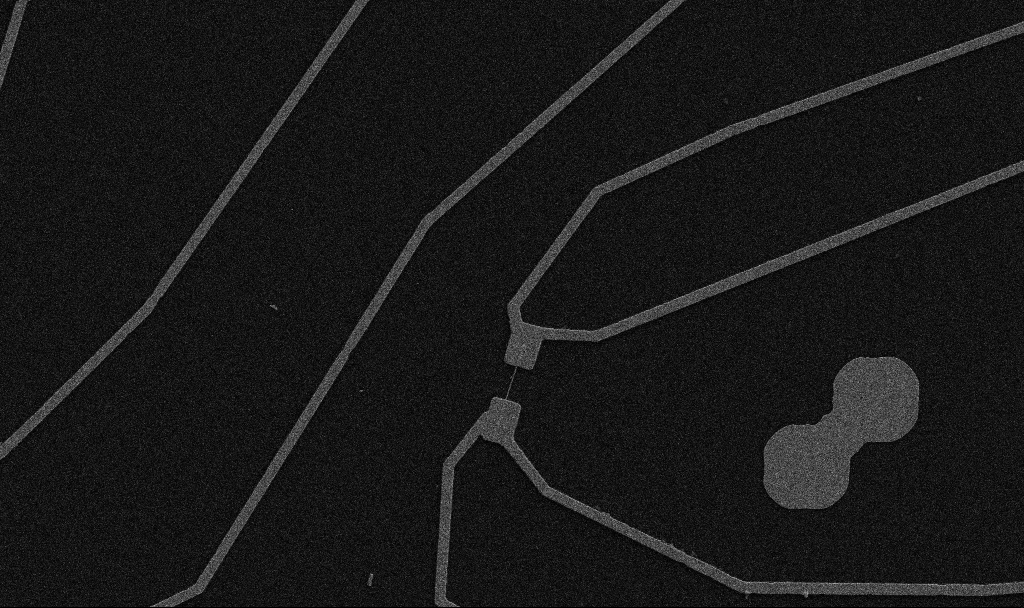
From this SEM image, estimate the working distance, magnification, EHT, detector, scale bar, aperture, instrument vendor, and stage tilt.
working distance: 10.7 mm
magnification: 5 K X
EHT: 5 kV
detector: SE2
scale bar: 10000 nm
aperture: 30 µm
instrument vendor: Zeiss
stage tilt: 0°